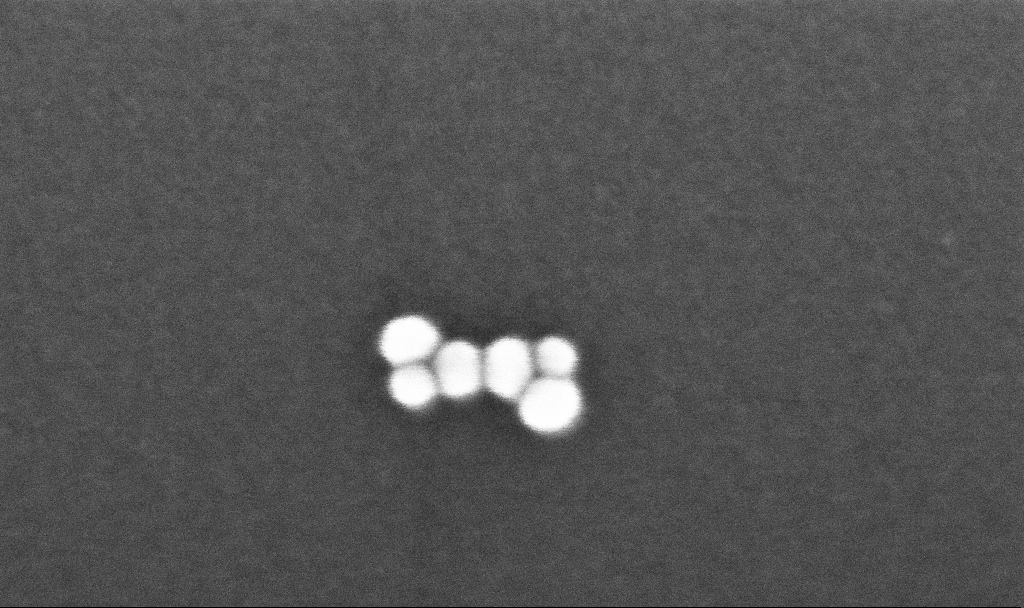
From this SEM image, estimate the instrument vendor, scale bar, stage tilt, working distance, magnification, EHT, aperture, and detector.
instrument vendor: Zeiss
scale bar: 20 nm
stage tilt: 0°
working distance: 3.3 mm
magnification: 860.89 K X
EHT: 10 kV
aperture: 30 µm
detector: InLens